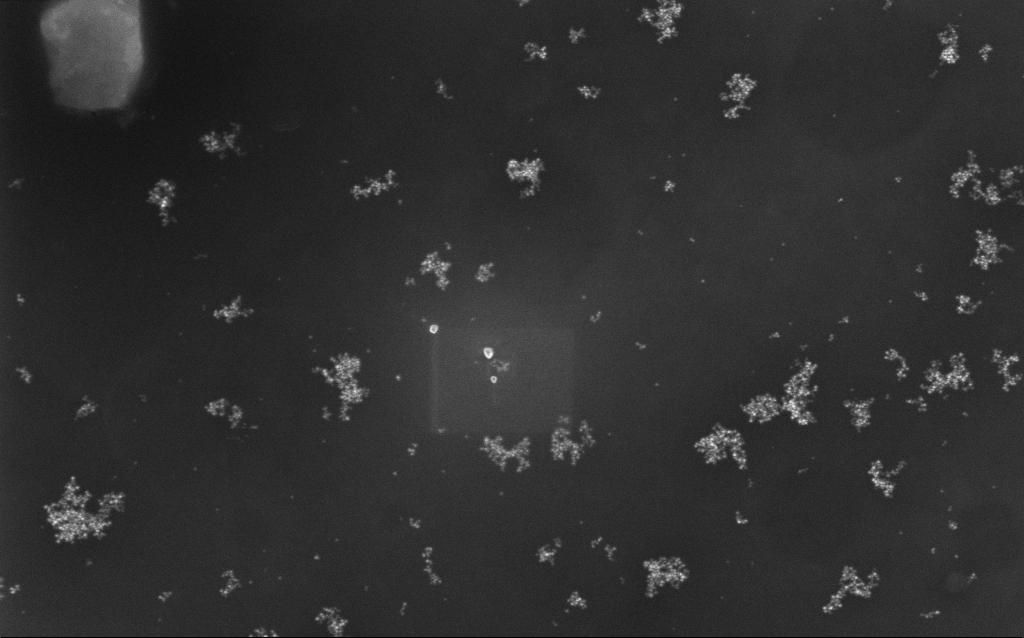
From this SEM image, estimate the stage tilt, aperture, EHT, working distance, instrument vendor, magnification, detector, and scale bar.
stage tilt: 0°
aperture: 30 µm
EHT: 10 kV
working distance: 7 mm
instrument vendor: Zeiss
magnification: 61.56 K X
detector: InLens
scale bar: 1000 nm